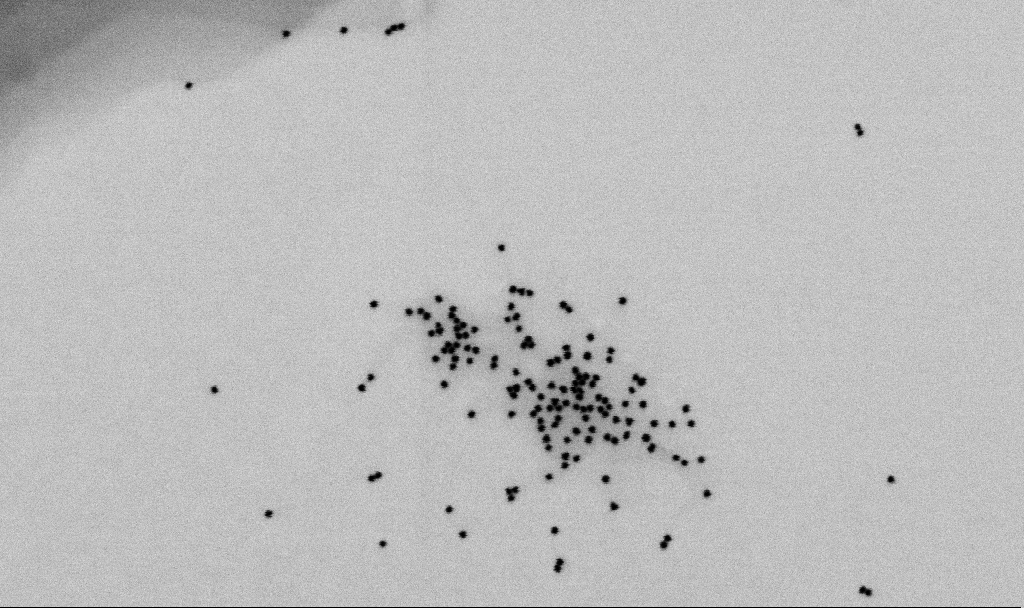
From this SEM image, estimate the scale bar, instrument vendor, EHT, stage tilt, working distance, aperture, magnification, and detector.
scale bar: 200 nm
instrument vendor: Zeiss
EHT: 2 kV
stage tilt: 0°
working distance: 6.5 mm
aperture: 30 µm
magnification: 121.42 K X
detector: SE2